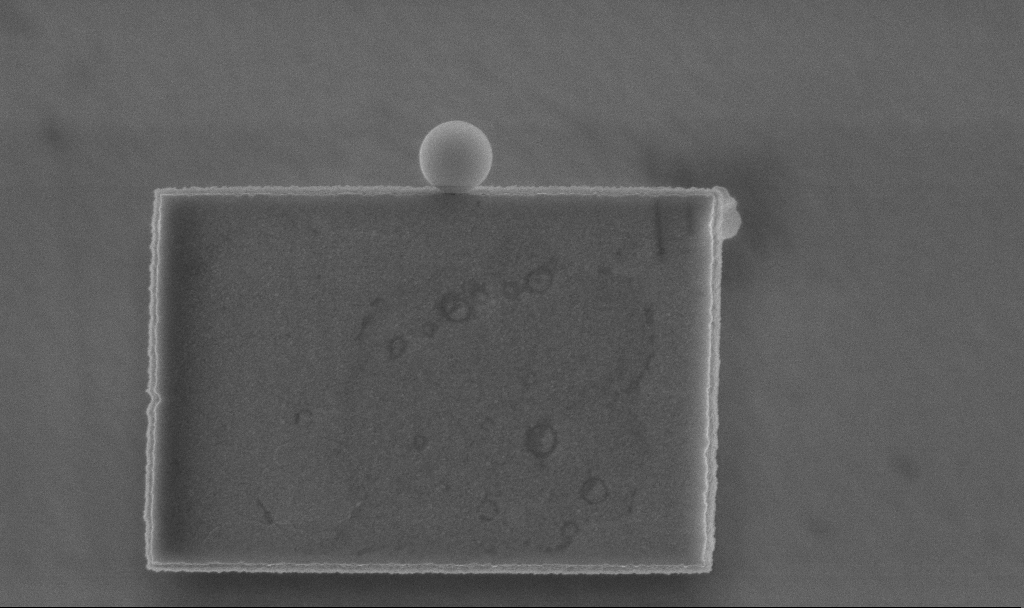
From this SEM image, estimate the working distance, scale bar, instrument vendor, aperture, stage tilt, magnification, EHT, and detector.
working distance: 3.3 mm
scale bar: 1000 nm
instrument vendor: Zeiss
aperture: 30 µm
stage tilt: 0°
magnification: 47.17 K X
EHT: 5 kV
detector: InLens